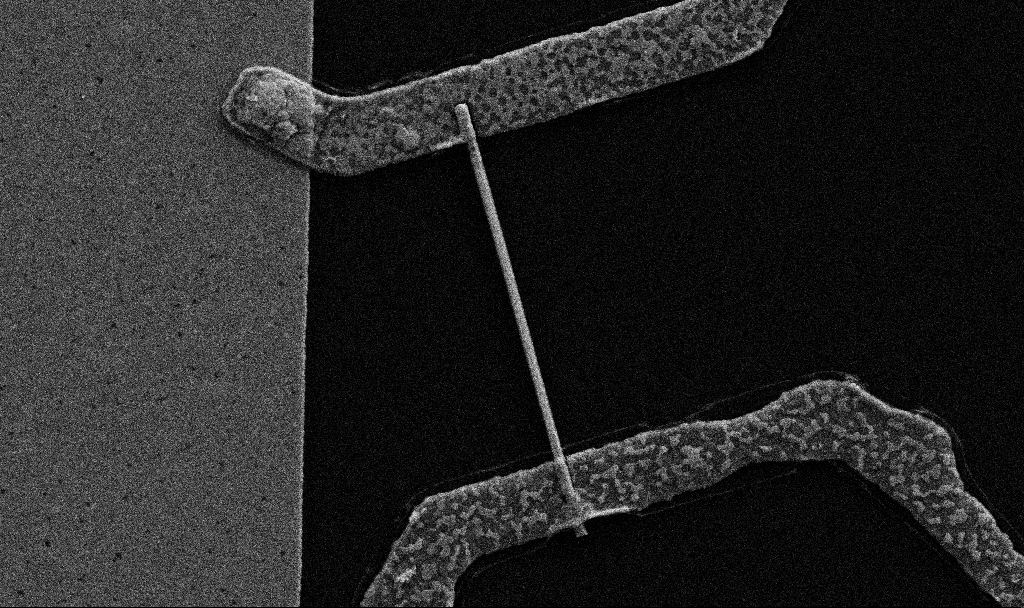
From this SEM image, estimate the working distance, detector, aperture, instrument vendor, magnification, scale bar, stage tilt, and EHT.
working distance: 6.7 mm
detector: SE2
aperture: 30 µm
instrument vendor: Zeiss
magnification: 30 K X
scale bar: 2000 nm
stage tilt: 0°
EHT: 5 kV